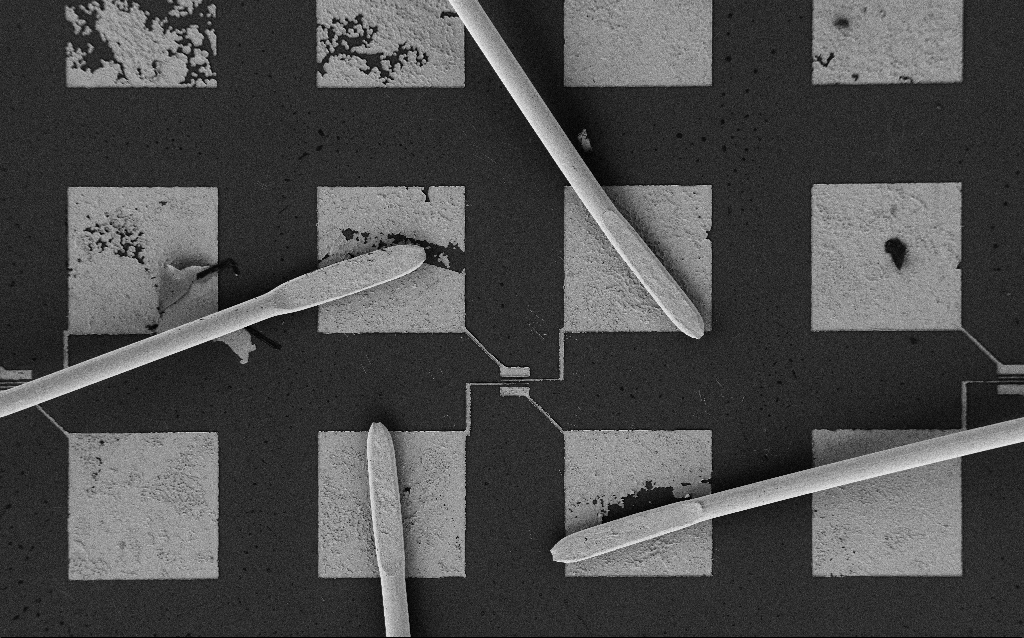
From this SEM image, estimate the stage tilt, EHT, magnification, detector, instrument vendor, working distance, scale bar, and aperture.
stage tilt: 0°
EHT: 2 kV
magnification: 0.362 K X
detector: SE2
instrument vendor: Zeiss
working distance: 8.1 mm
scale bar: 100000 nm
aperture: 30 µm